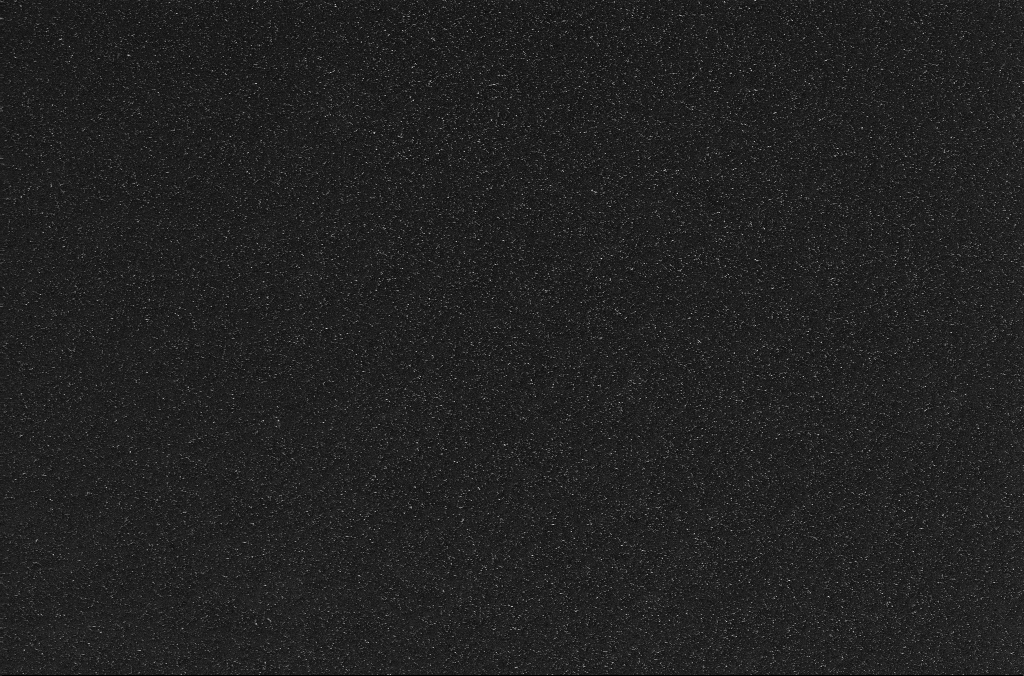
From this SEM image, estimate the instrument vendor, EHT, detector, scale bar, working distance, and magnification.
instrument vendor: Zeiss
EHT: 10 kV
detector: InLens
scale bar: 10000 nm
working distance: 3.2 mm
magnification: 5.44 K X